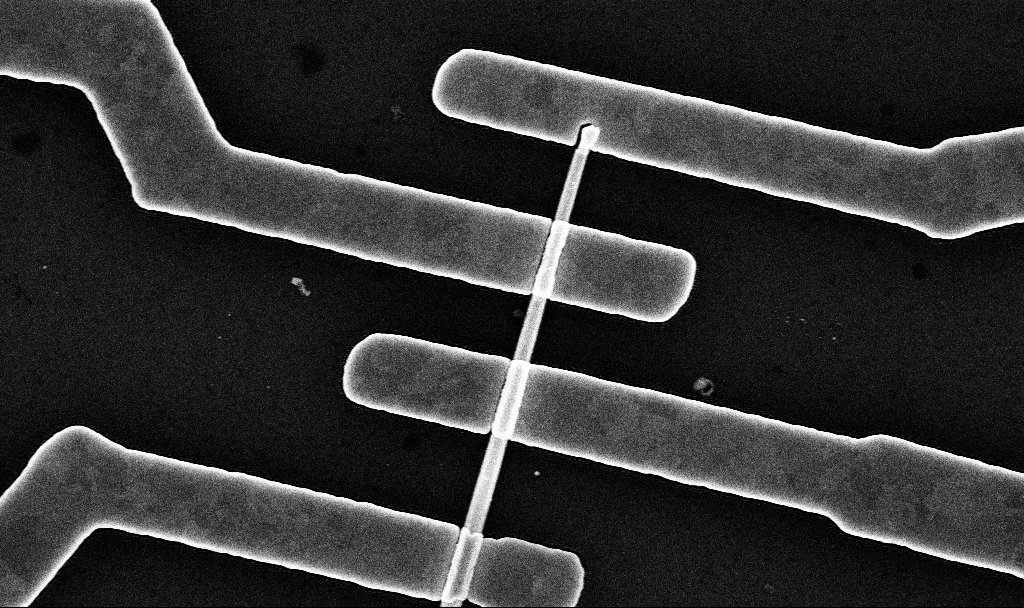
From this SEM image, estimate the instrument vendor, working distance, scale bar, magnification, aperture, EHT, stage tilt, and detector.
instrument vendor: Zeiss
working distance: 7.6 mm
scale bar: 1000 nm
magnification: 38.94 K X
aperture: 30 µm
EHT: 10 kV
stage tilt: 0°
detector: InLens